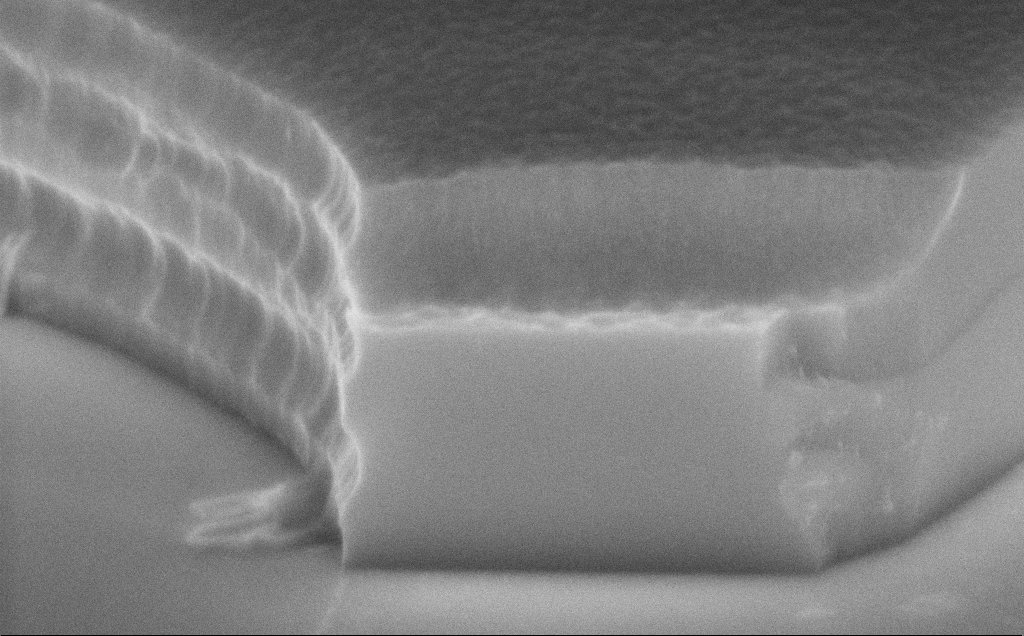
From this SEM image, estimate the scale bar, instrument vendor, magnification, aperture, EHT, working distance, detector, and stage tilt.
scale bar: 1000 nm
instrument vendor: Zeiss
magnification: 57.89 K X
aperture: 30 µm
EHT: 8 kV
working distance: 12 mm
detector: InLens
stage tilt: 70°